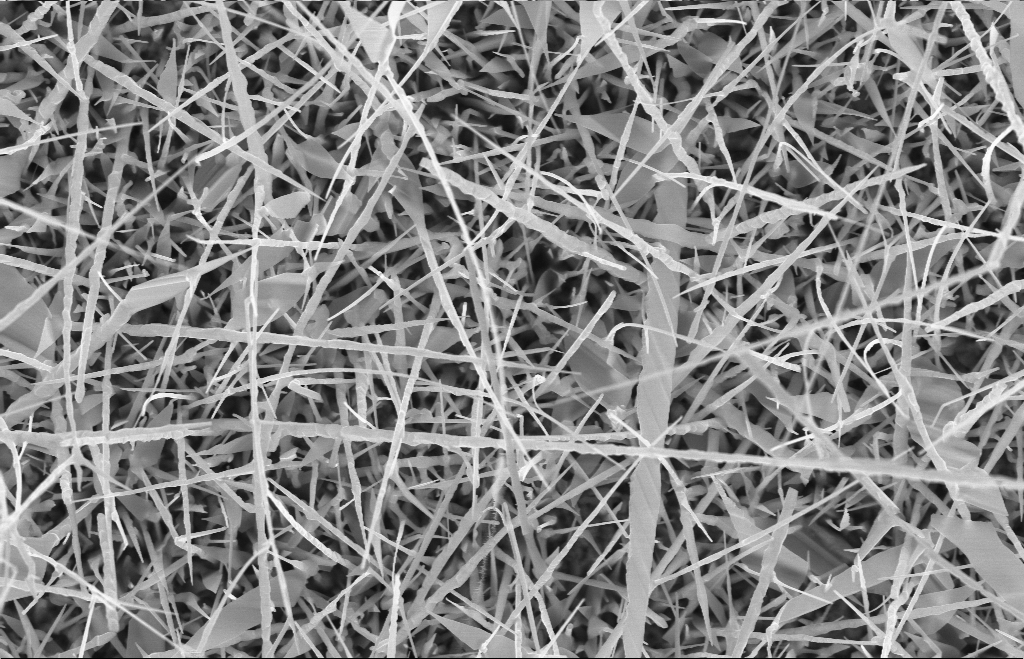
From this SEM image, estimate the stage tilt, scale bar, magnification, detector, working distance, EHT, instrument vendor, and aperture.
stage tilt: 0°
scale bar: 2000 nm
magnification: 20 K X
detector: InLens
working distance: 9 mm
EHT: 10 kV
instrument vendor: Zeiss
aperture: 30 µm